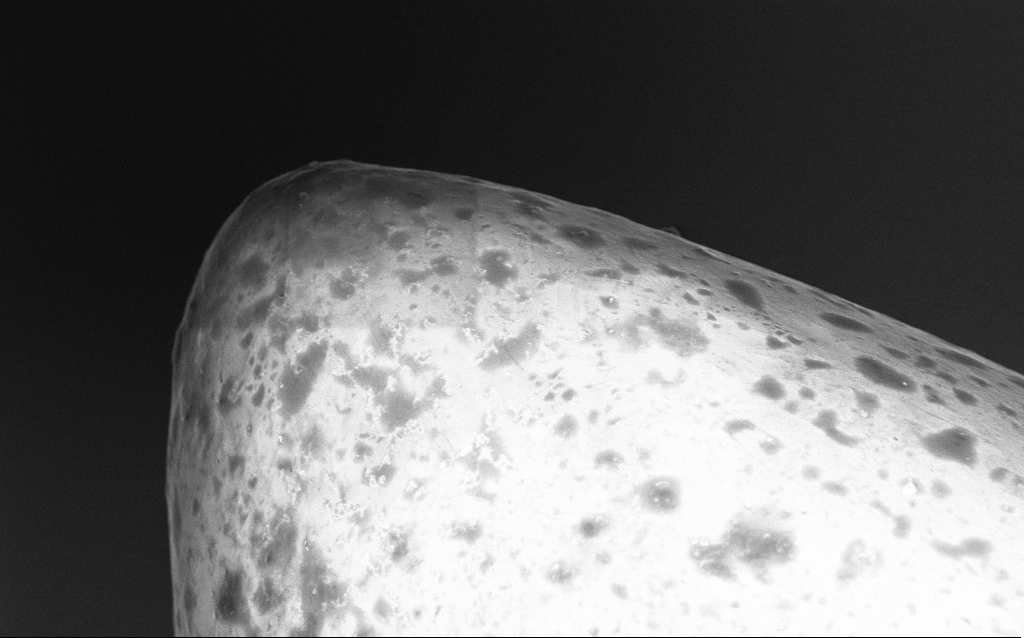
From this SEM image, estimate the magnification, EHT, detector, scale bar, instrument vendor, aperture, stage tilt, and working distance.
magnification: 19.21 K X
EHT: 5 kV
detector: InLens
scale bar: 1000 nm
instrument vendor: Zeiss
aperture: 30 µm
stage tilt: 0°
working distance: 3.1 mm